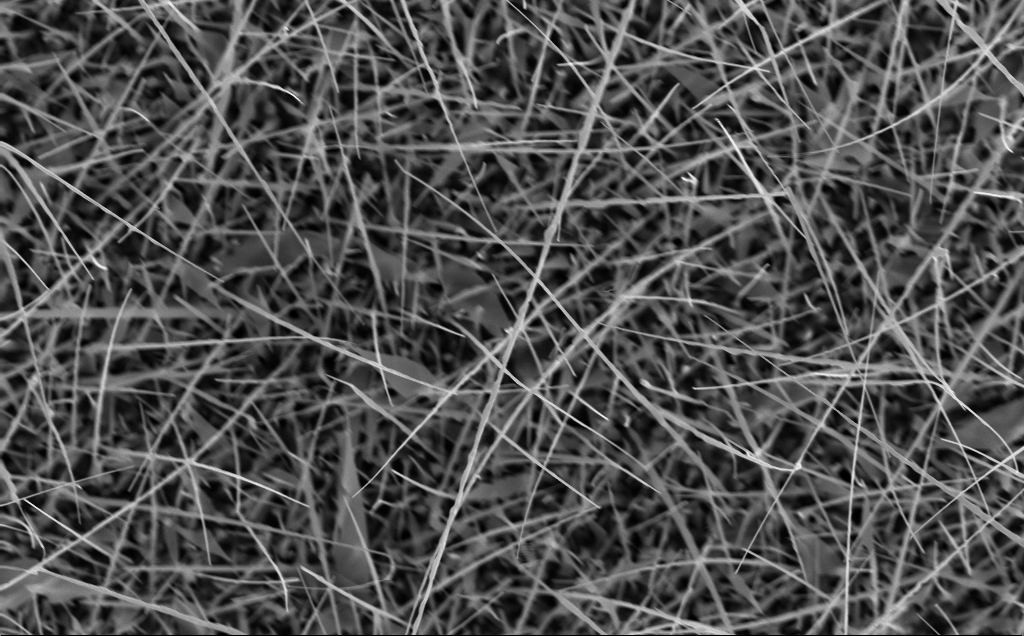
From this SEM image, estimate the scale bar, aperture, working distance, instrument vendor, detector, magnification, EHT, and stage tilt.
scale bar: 1000 nm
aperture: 30 µm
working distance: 5 mm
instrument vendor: Zeiss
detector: InLens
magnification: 20 K X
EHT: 10 kV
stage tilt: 0°